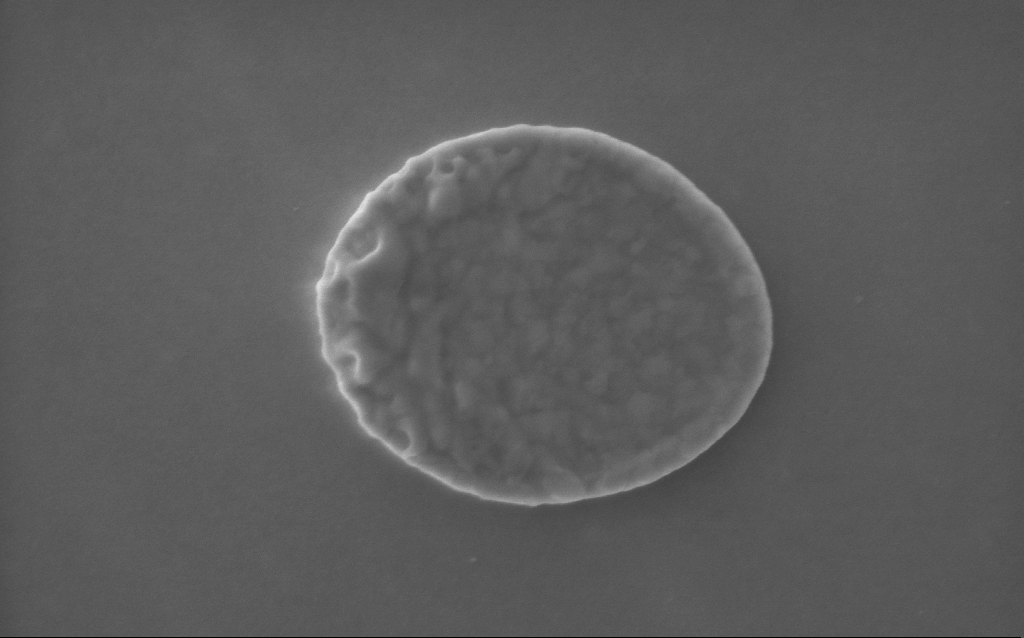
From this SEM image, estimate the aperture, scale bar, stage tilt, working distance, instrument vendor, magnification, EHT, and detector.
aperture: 30 µm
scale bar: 200 nm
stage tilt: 0°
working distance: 2 mm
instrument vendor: Zeiss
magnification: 92.45 K X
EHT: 5 kV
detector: InLens